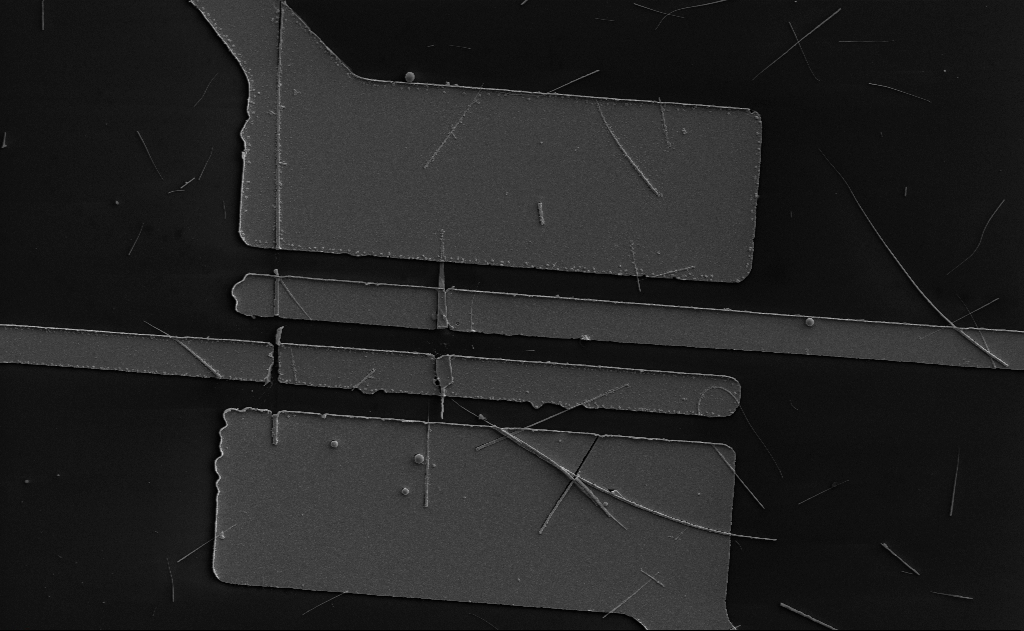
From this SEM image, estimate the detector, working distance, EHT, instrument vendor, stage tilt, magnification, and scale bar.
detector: SE2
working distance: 15 mm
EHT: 5 kV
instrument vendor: Zeiss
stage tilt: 0°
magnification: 6.28 K X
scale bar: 10000 nm